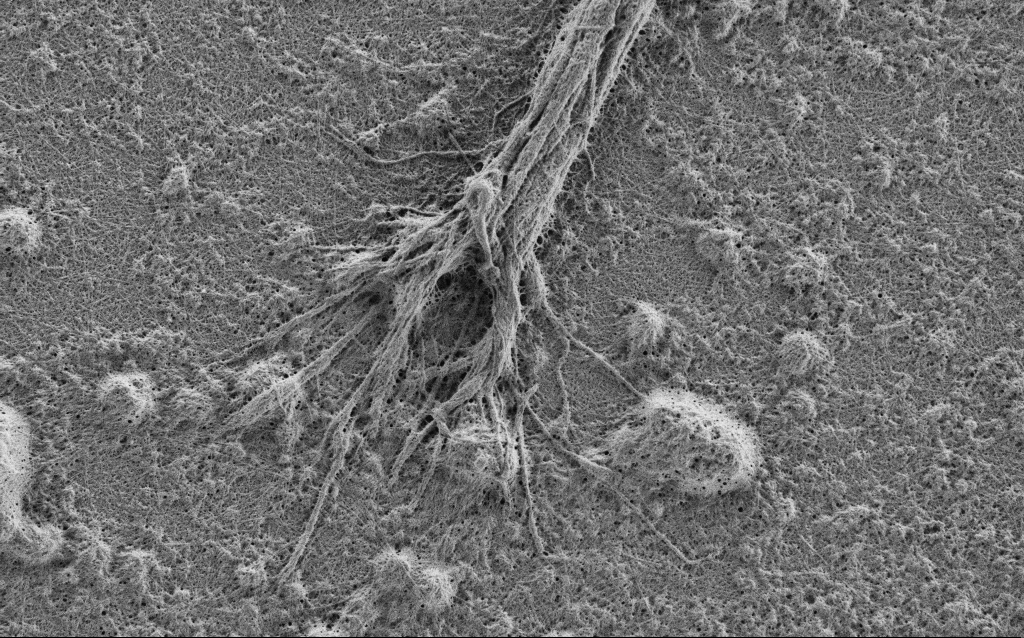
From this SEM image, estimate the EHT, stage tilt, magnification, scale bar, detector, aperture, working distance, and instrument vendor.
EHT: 0.9 kV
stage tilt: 0°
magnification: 10 K X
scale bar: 2000 nm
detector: SE2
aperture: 30 µm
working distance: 4 mm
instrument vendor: Zeiss